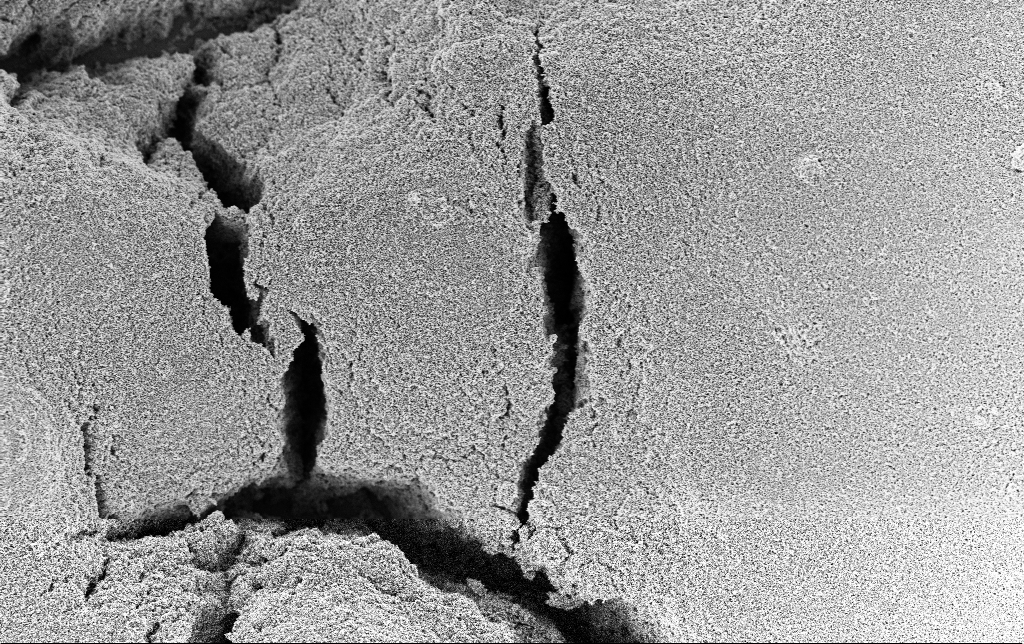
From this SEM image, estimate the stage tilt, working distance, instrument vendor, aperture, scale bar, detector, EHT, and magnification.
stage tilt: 0°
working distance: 2.4 mm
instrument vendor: Zeiss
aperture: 30 µm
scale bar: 10000 nm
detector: InLens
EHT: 3 kV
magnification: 5 K X